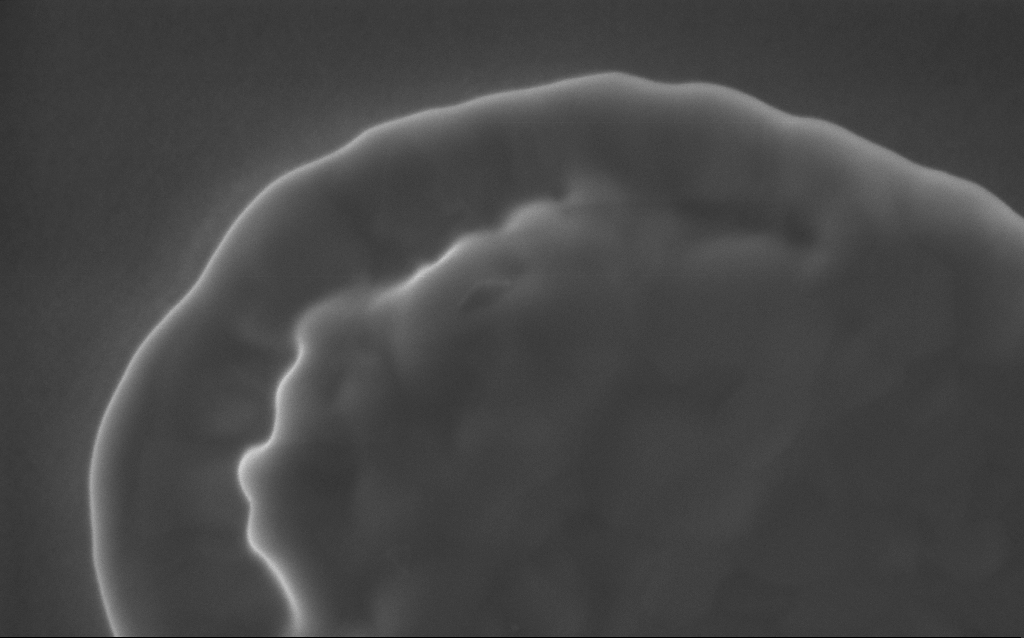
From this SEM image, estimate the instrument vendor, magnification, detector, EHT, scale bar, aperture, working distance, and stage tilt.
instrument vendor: Zeiss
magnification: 217 K X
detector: InLens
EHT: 5 kV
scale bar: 200 nm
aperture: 30 µm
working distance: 3 mm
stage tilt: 0°